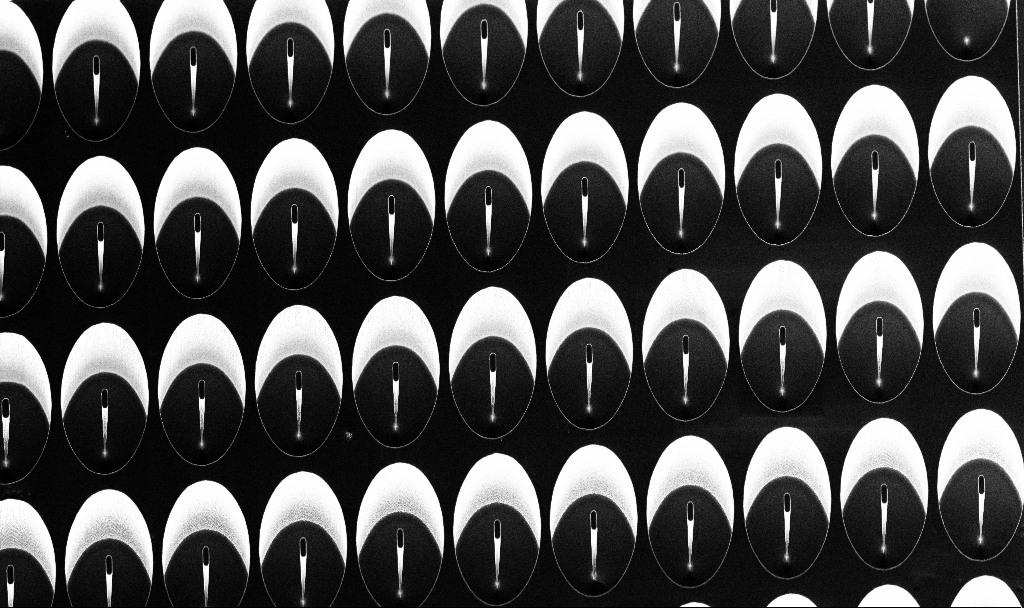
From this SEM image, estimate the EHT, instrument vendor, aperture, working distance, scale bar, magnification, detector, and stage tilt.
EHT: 5 kV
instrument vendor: Zeiss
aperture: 30 µm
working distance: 6.8 mm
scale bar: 20000 nm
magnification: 1.08 K X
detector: InLens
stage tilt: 29.2°